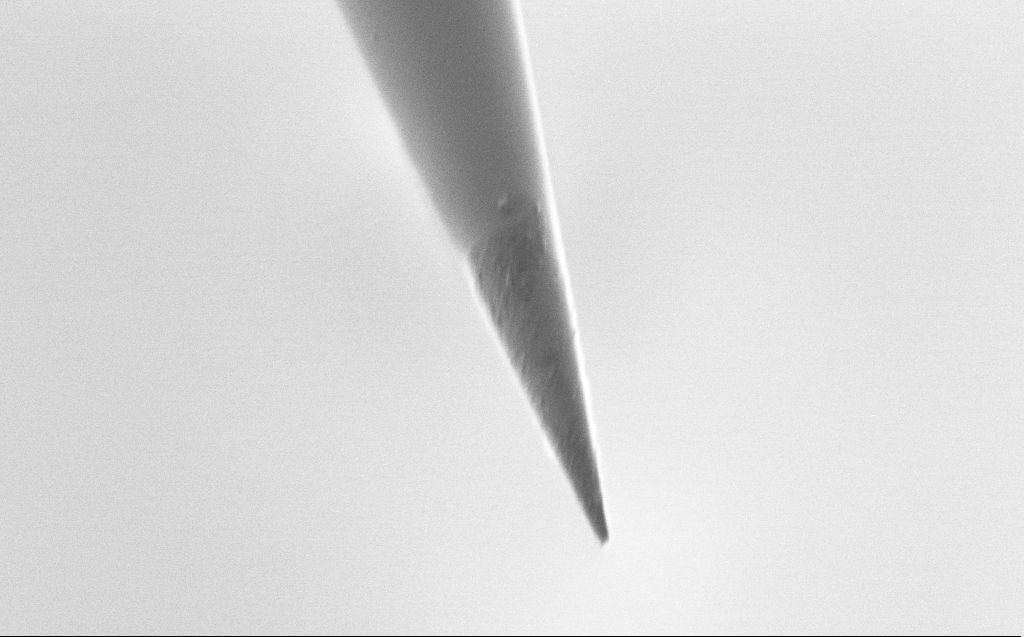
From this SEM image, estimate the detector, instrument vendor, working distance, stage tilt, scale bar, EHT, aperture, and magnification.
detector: SE2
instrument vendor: Zeiss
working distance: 5 mm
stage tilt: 39.3°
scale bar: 1000 nm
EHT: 0.8 kV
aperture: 30 µm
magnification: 50 K X